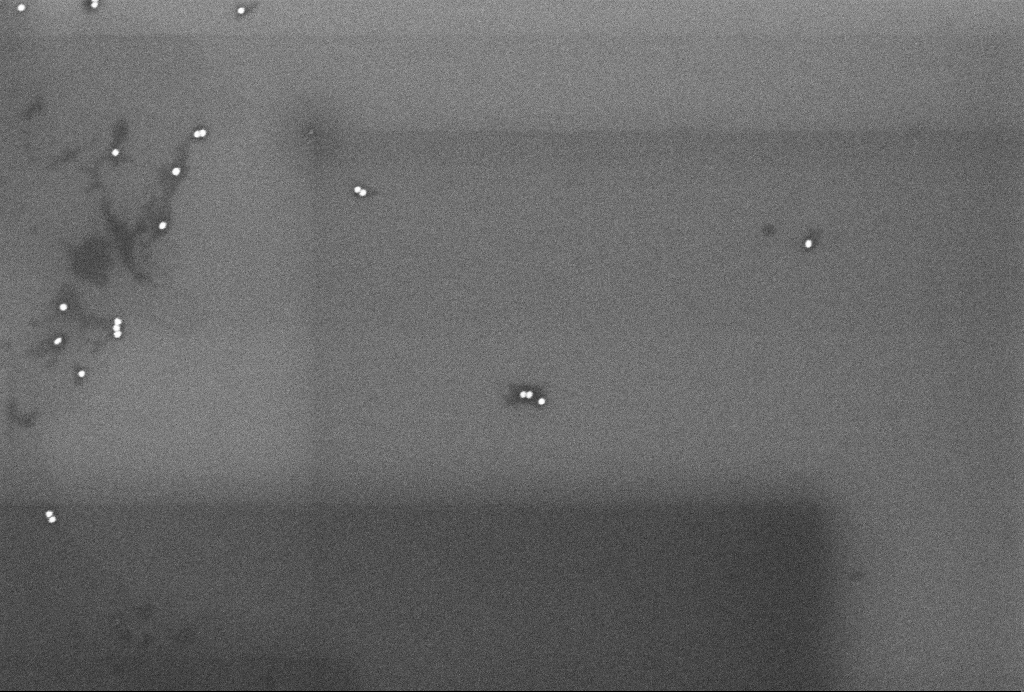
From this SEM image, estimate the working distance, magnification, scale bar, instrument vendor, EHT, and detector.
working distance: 3.2 mm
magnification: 95 K X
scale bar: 200 nm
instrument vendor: Zeiss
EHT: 4 kV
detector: InLens